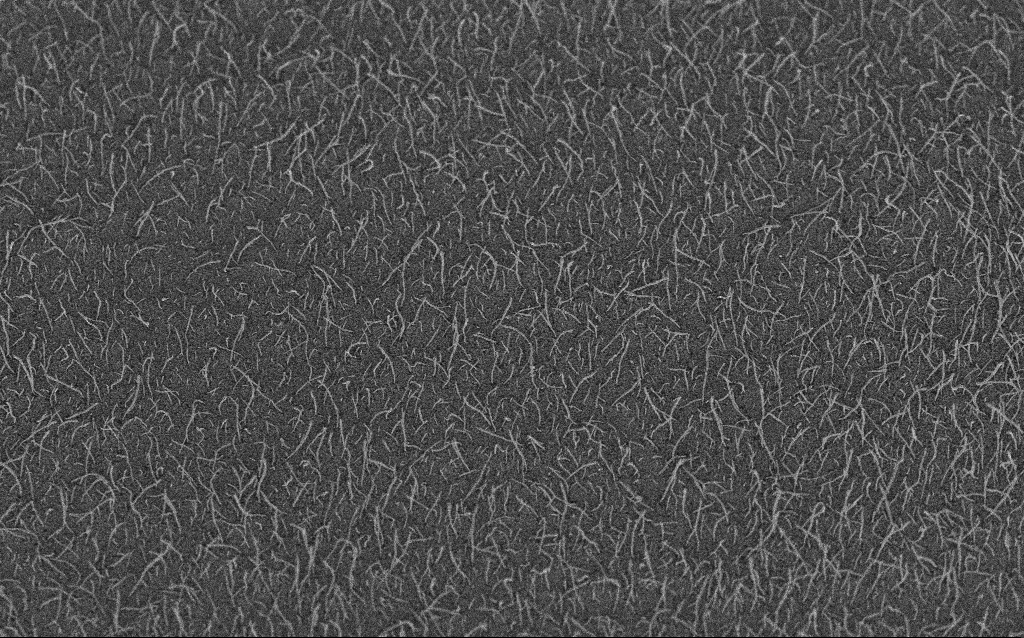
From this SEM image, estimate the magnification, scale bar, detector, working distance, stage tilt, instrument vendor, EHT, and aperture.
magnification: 10 K X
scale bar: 2000 nm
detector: InLens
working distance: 8.7 mm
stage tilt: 45°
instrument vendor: Zeiss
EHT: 5 kV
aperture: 30 µm